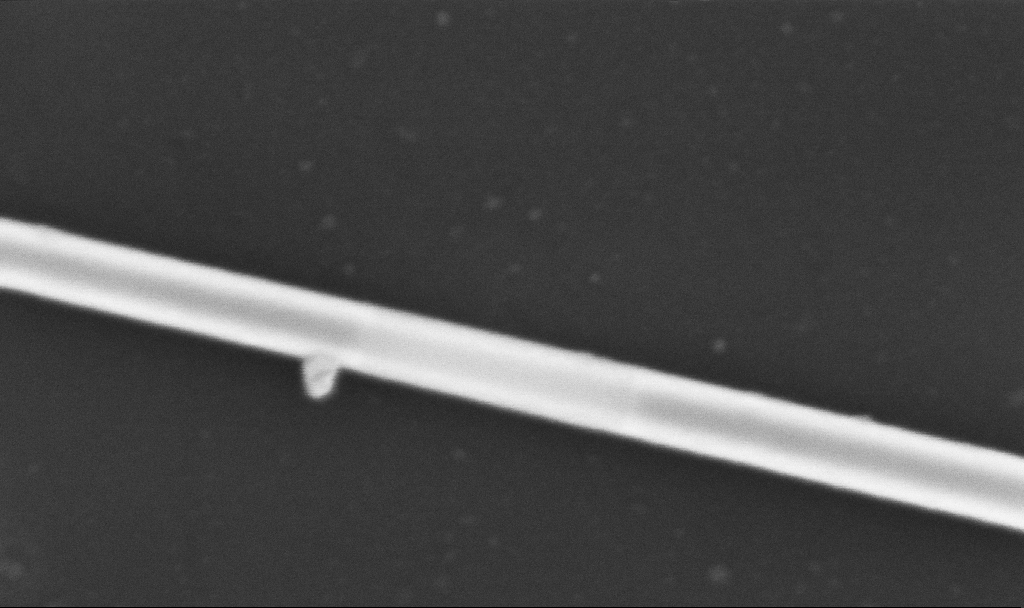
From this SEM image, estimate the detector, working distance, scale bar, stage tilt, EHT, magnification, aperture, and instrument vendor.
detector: InLens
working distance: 6.7 mm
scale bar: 100 nm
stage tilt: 0°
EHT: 5 kV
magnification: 300 K X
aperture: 30 µm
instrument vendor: Zeiss